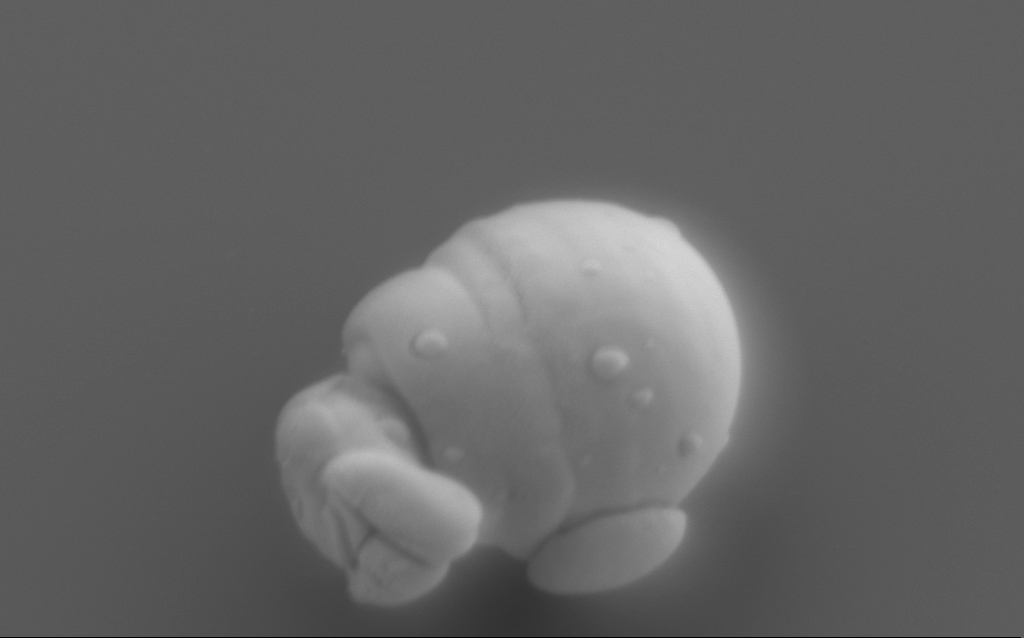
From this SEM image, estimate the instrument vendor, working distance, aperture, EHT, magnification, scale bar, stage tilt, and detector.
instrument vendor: Zeiss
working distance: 6 mm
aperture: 120 µm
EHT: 4 kV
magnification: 52.23 K X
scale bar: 1000 nm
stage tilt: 22°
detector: SE2